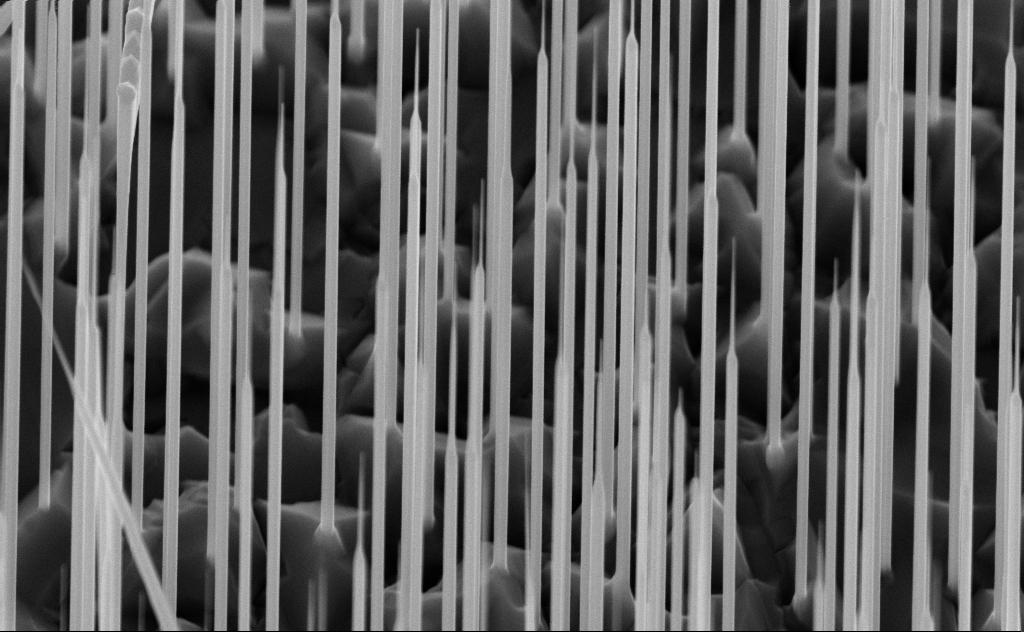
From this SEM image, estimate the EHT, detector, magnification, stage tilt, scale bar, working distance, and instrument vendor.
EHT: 10 kV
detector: InLens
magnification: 40 K X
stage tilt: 45°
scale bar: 1000 nm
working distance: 6 mm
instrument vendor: Zeiss